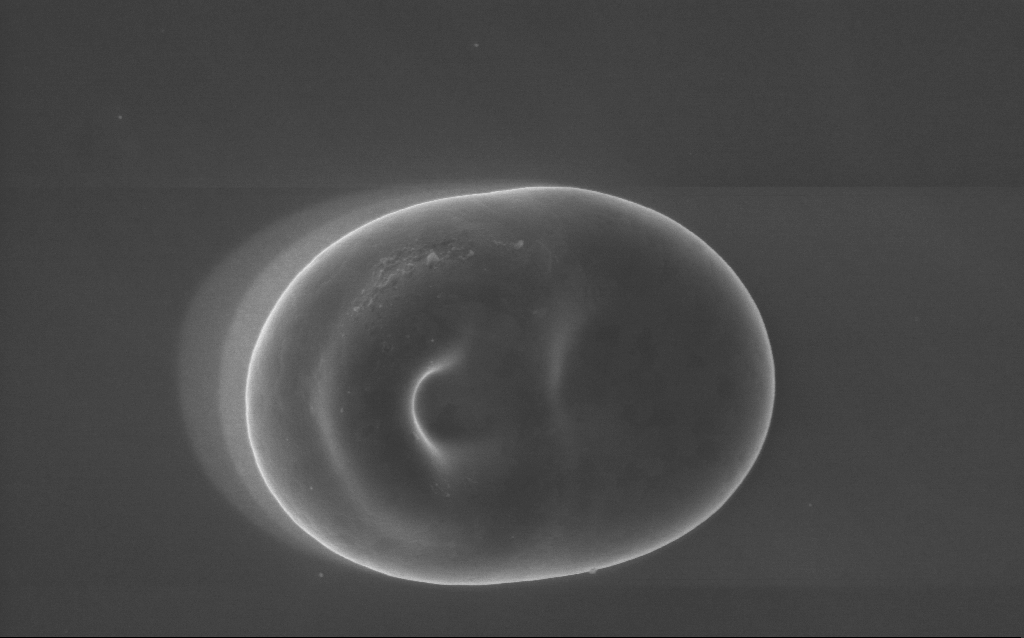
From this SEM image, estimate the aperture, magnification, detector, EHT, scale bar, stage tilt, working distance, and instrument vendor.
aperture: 30 µm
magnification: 38 K X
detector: InLens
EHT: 5 kV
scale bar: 1000 nm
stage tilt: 0°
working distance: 3 mm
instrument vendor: Zeiss